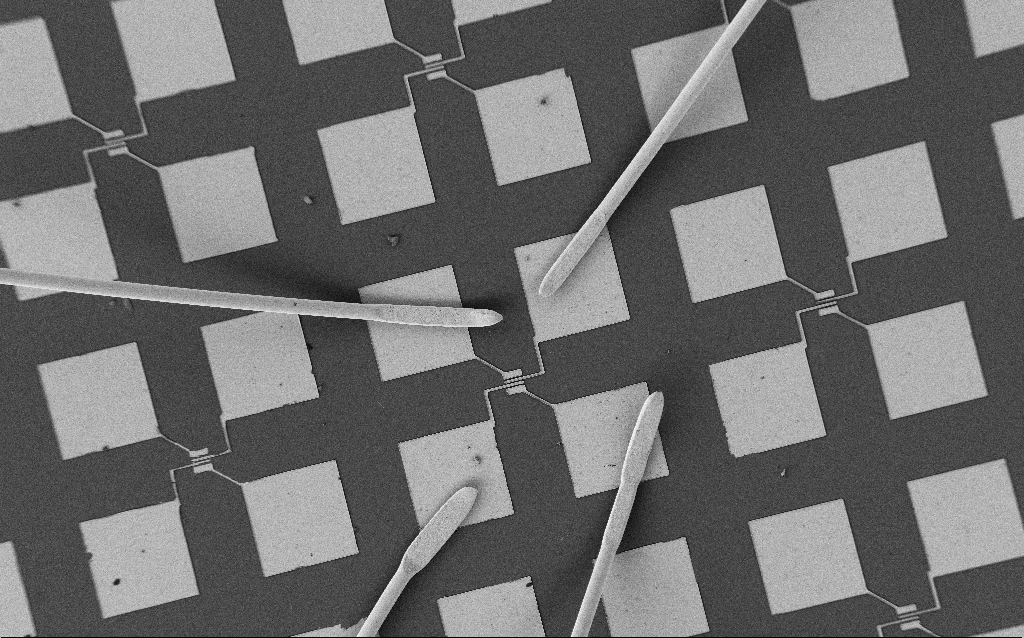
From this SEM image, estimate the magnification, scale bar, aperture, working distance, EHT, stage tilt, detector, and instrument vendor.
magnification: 0.235 K X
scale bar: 100000 nm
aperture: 30 µm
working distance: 7.7 mm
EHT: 2 kV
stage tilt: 0°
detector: SE2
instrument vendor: Zeiss